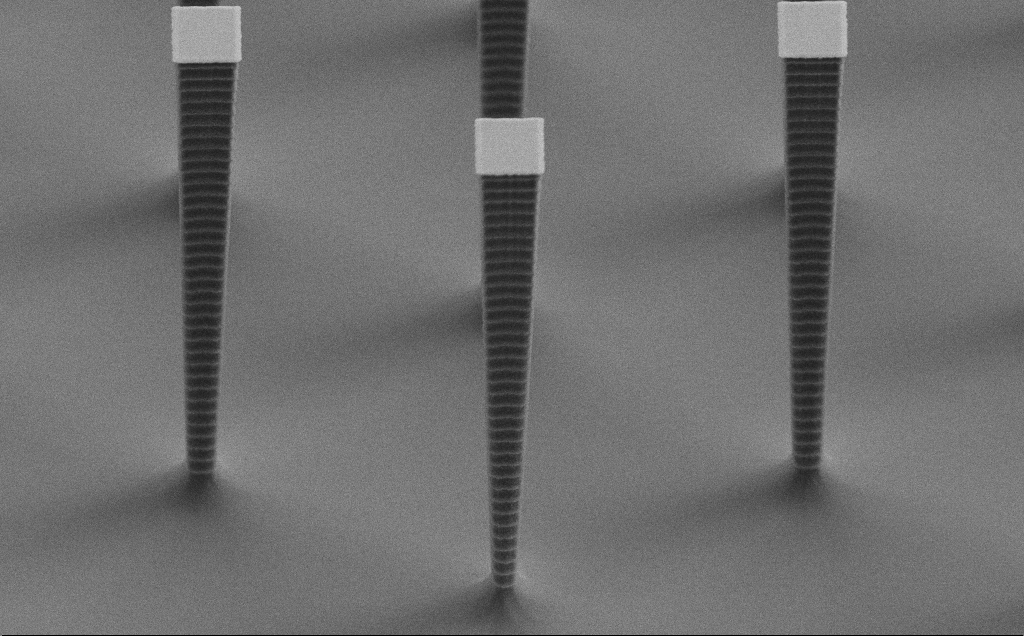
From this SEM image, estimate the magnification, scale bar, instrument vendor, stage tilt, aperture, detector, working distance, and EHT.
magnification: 12.28 K X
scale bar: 1000 nm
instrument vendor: Zeiss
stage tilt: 60°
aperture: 30 µm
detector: SE2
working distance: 7 mm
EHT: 3 kV